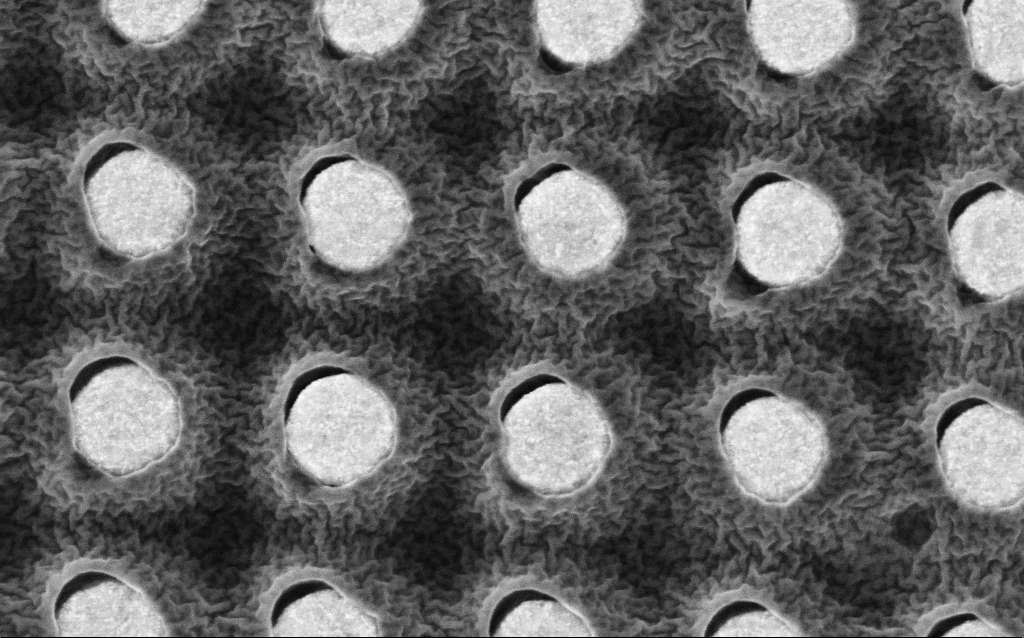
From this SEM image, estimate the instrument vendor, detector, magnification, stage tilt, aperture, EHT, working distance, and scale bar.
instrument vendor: Zeiss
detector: InLens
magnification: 160.73 K X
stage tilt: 0°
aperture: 30 µm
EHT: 2 kV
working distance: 3.1 mm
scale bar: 200 nm